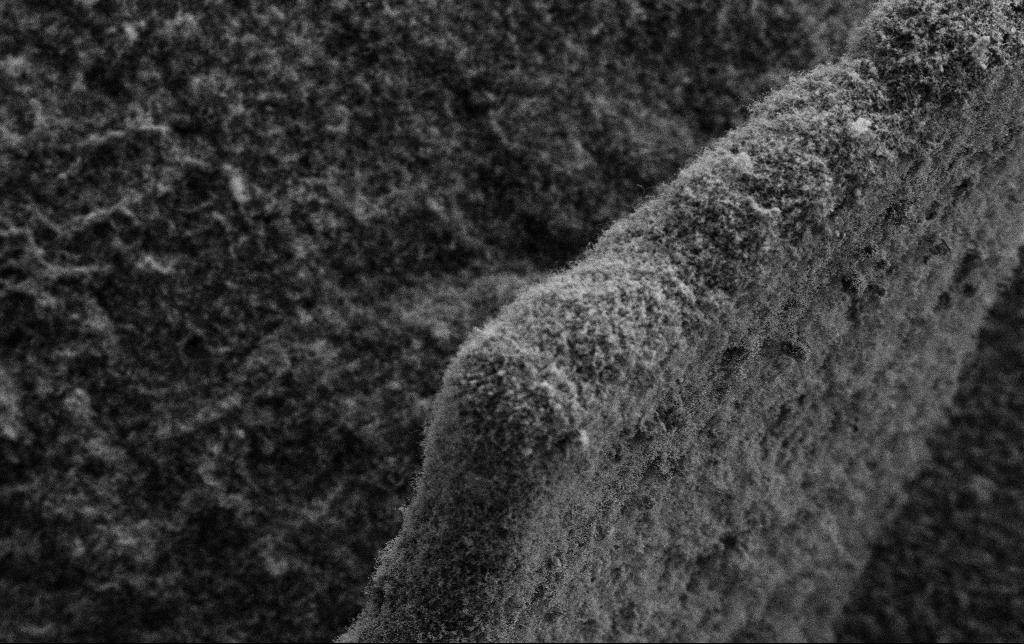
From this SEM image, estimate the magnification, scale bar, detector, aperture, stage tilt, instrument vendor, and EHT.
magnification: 1.5 K X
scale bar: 10000 nm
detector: SE2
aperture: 30 µm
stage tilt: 0°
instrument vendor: Zeiss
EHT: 2 kV